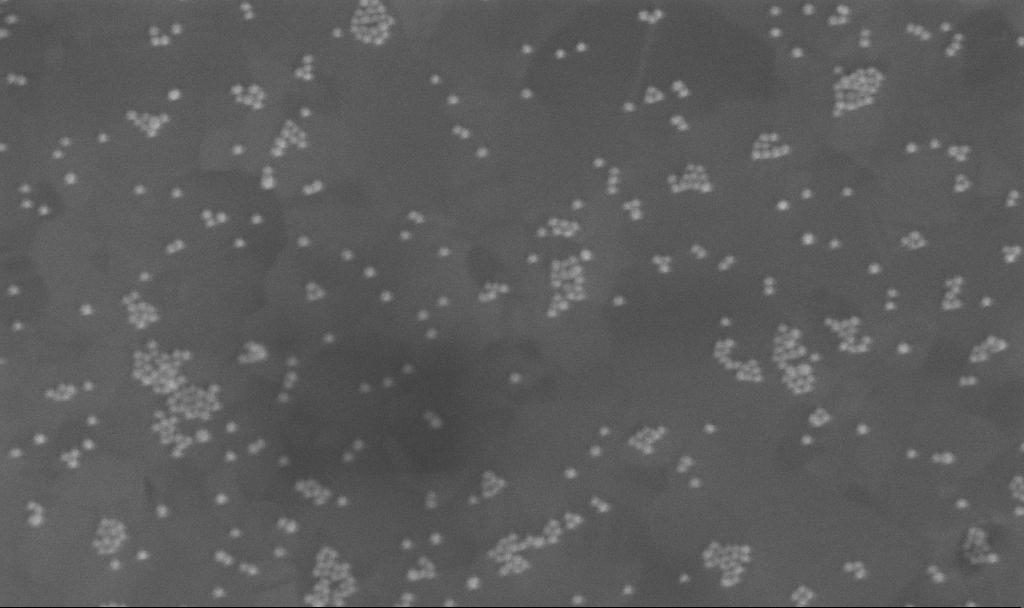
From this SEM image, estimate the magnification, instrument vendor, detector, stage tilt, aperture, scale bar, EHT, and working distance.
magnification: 198.62 K X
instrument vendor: Zeiss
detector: InLens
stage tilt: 0°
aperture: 30 µm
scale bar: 200 nm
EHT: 10 kV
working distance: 3.9 mm